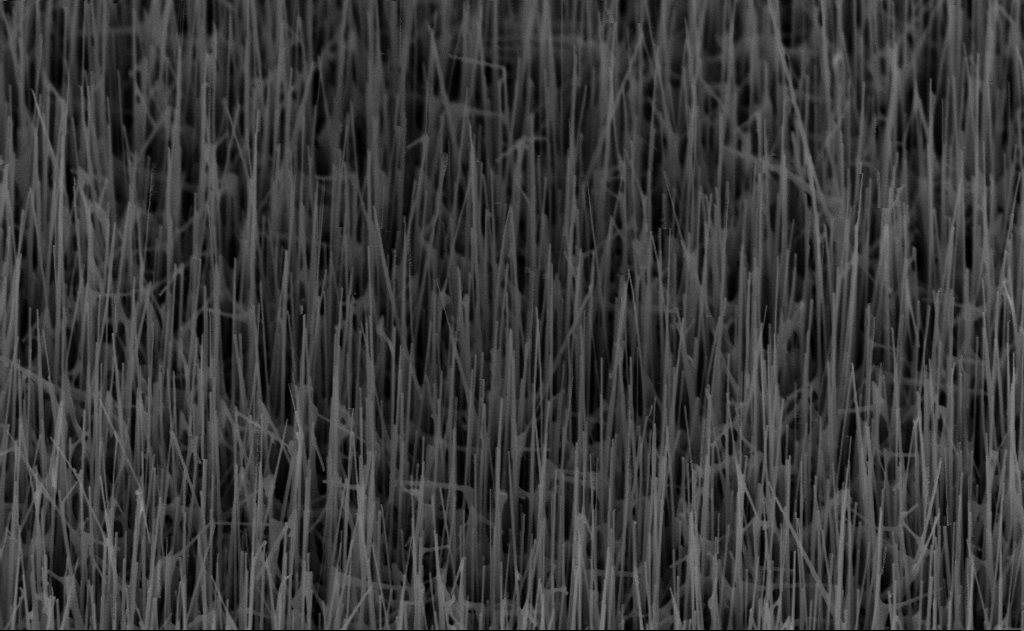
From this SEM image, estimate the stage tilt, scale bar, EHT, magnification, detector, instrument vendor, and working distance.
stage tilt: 45°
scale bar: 1000 nm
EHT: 10 kV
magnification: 40 K X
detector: InLens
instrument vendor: Zeiss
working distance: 6 mm